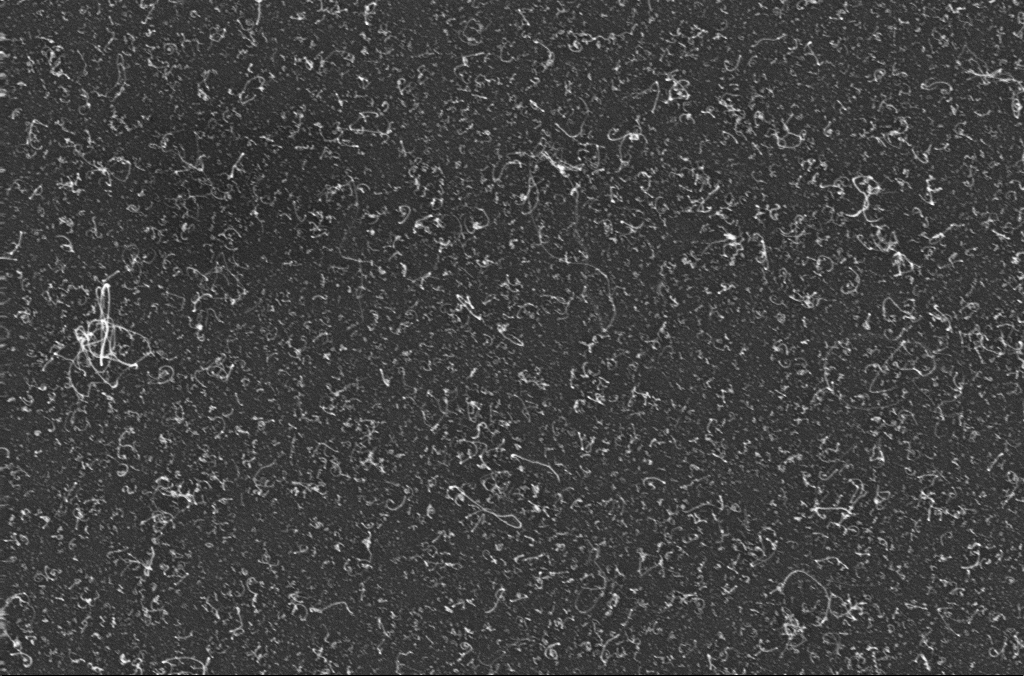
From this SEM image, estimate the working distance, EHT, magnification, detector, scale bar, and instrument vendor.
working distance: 3.3 mm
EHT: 10 kV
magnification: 50 K X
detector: InLens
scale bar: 1000 nm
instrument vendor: Zeiss